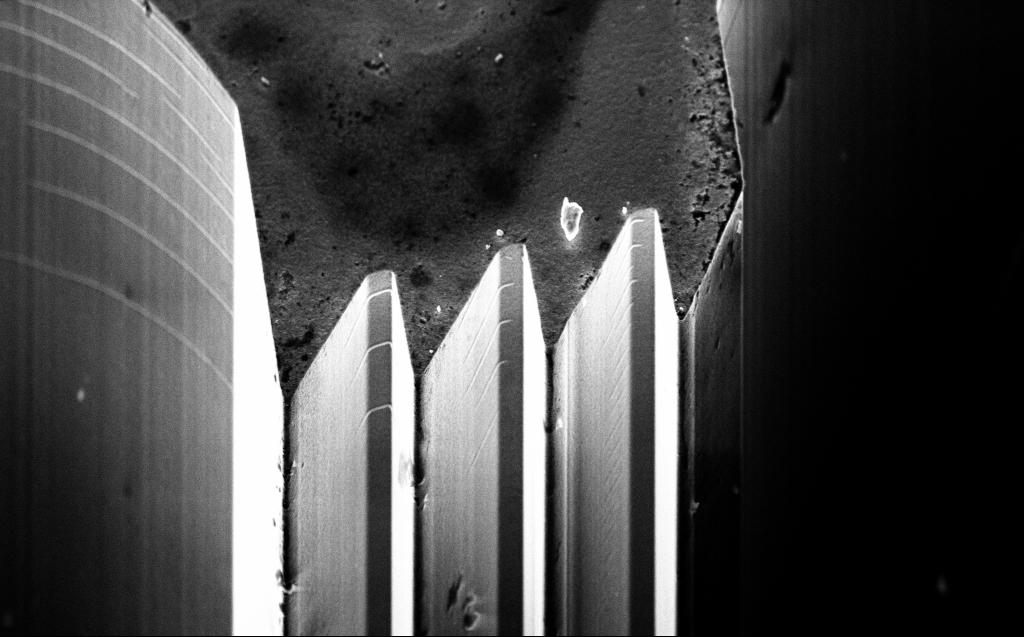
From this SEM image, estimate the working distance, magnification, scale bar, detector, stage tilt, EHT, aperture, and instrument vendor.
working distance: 9 mm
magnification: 4.33 K X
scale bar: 10000 nm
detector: InLens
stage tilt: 45°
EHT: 10 kV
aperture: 30 µm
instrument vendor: Zeiss